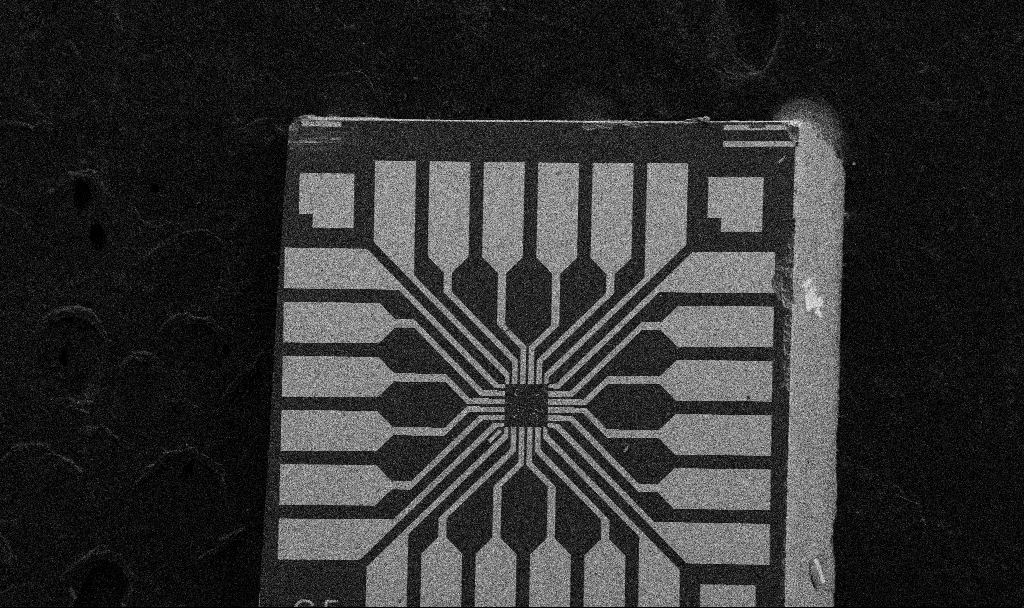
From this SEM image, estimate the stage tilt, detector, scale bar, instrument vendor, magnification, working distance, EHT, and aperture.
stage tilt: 0°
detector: SE2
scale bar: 200000 nm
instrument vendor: Zeiss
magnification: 0.1 K X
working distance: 10.7 mm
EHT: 5 kV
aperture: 30 µm